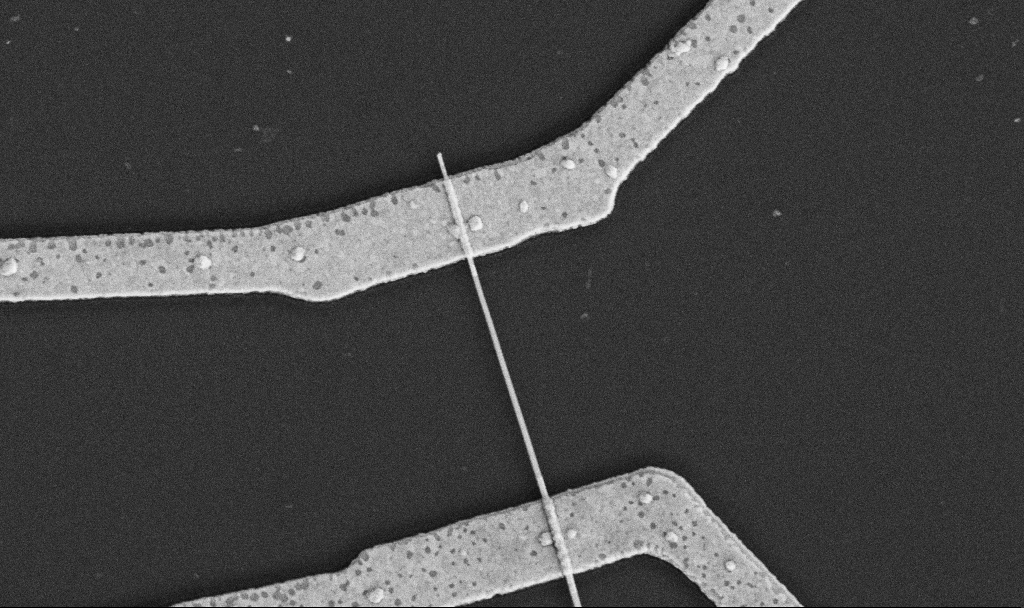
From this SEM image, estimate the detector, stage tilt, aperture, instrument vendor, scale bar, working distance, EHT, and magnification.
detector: SE2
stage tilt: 0°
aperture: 30 µm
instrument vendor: Zeiss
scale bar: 1000 nm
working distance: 10.6 mm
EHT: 5 kV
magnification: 30 K X